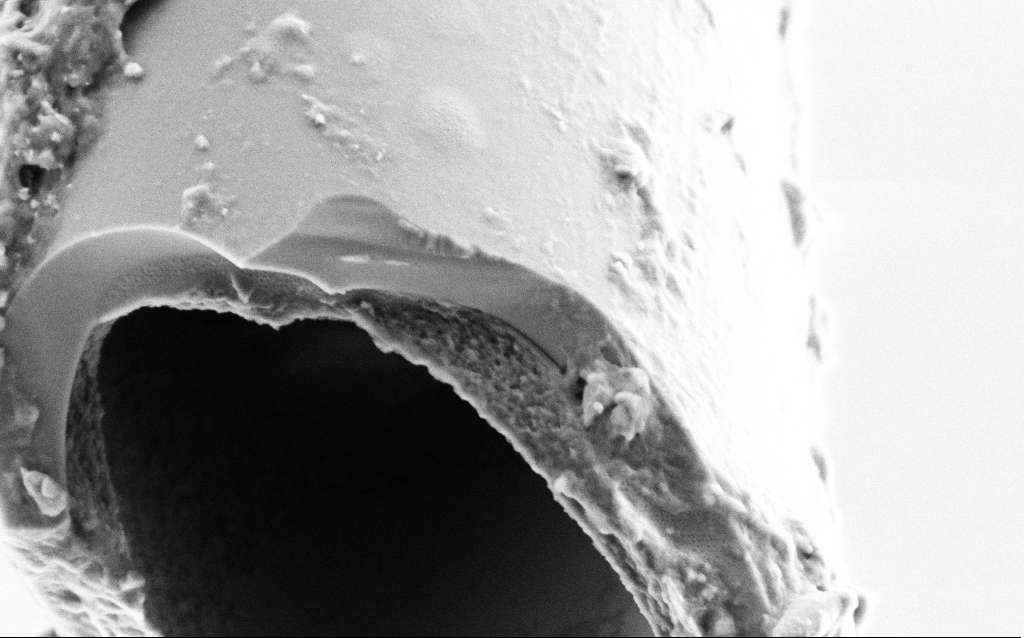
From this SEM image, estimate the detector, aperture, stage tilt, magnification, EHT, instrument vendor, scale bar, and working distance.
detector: SE2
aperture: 30 µm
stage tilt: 45°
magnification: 50 K X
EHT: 2 kV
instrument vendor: Zeiss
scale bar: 1000 nm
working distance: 6 mm